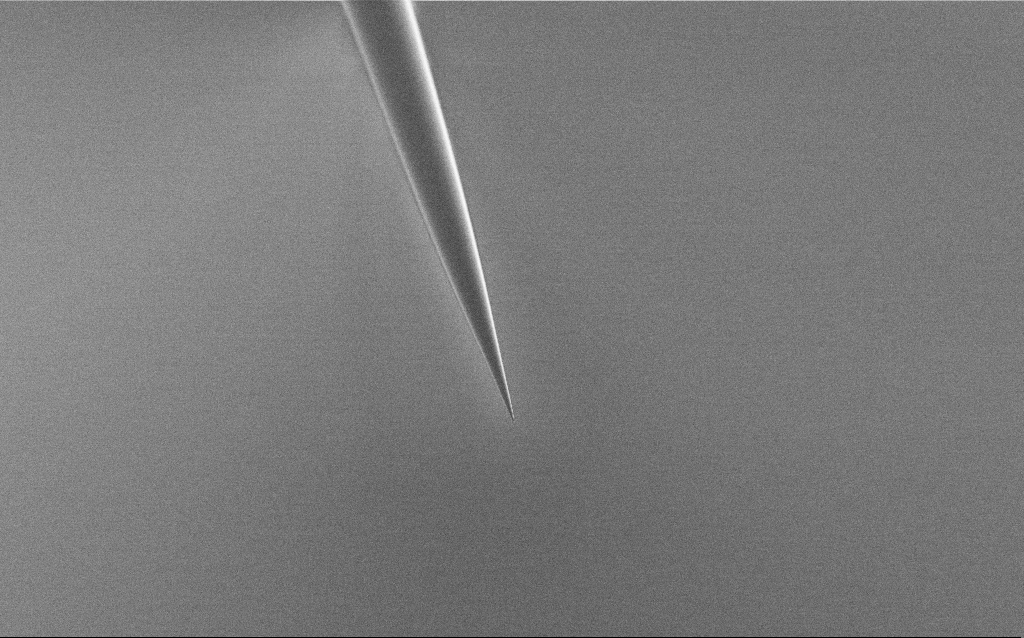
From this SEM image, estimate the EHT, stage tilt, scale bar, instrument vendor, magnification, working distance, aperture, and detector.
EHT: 1 kV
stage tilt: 45°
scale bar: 20000 nm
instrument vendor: Zeiss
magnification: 1 K X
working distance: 7 mm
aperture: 30 µm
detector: SE2